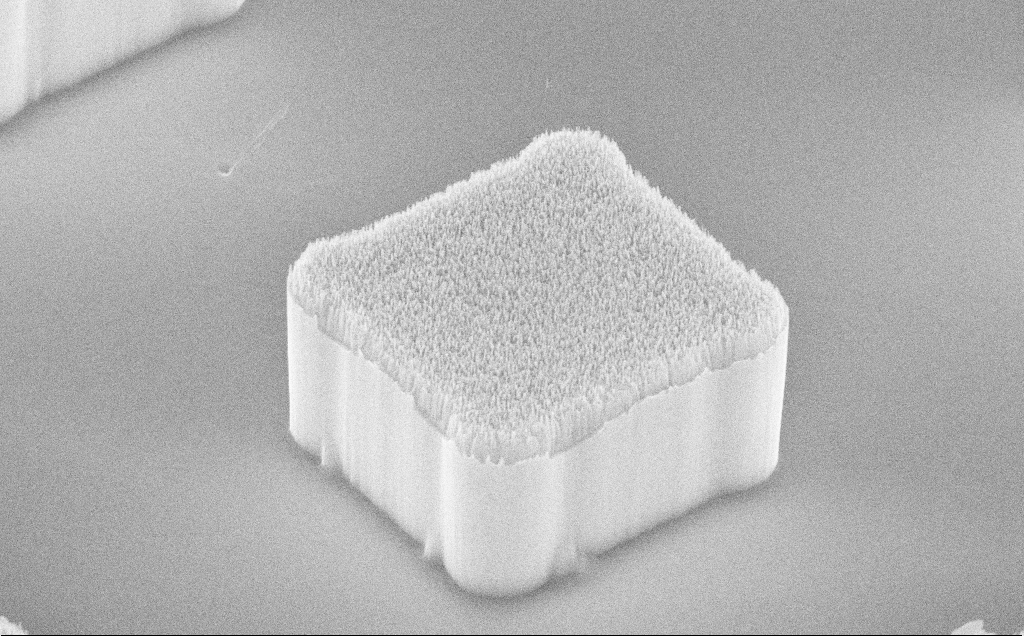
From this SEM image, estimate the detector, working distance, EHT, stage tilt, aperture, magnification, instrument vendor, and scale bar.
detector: InLens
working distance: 13 mm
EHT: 10 kV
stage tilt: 50.6°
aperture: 30 µm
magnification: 16.74 K X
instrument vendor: Zeiss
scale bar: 2000 nm